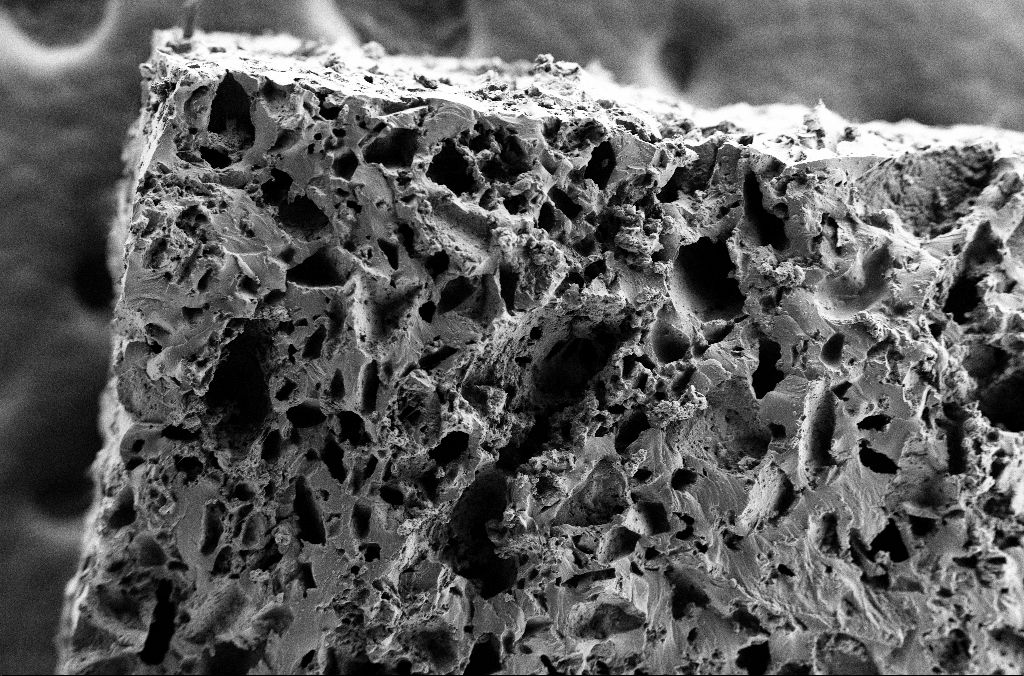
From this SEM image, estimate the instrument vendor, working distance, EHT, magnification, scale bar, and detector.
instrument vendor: Zeiss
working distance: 3 mm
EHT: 2 kV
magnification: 0.243 K X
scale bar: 100000 nm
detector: SE2